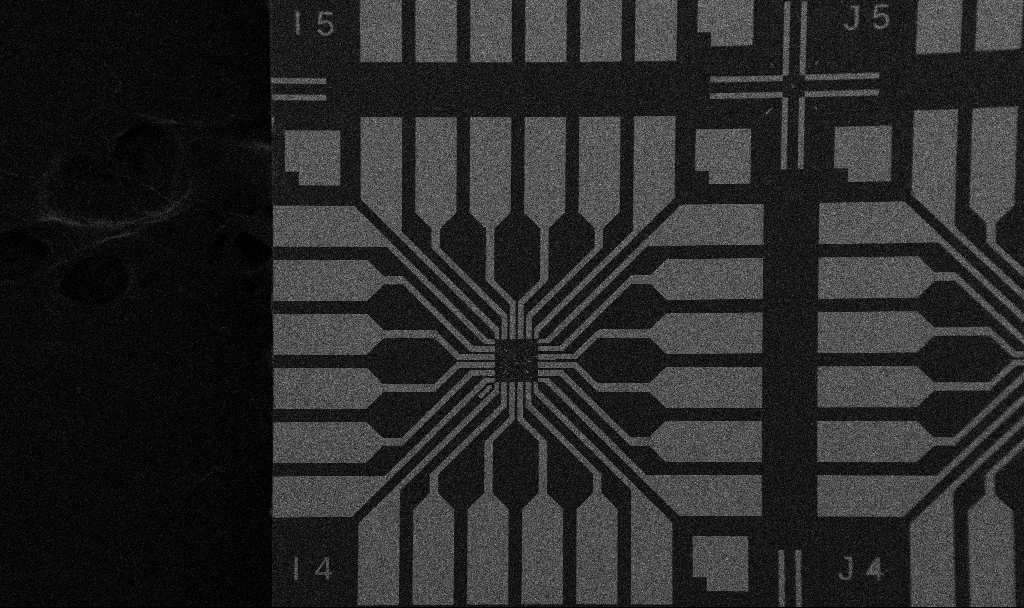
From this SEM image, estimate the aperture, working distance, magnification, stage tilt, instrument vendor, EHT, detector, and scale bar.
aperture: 30 µm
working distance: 10.7 mm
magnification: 0.1 K X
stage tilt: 0°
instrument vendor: Zeiss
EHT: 5 kV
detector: SE2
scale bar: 200000 nm